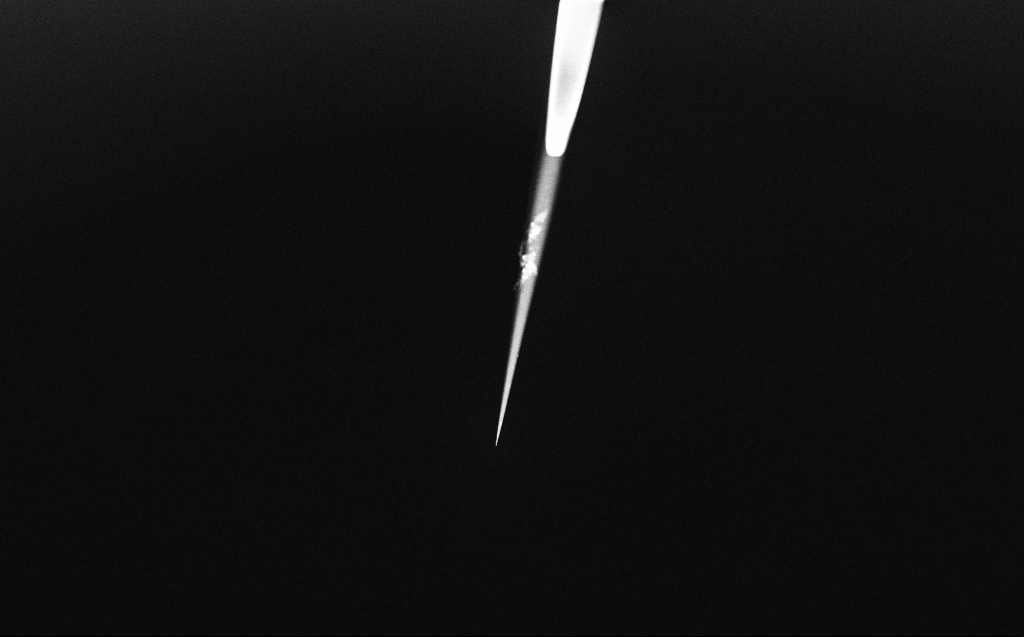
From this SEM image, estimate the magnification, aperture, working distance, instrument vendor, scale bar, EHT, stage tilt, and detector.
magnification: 1 K X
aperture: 30 µm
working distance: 4 mm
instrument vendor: Zeiss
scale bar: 20000 nm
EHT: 2 kV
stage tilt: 45°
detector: InLens